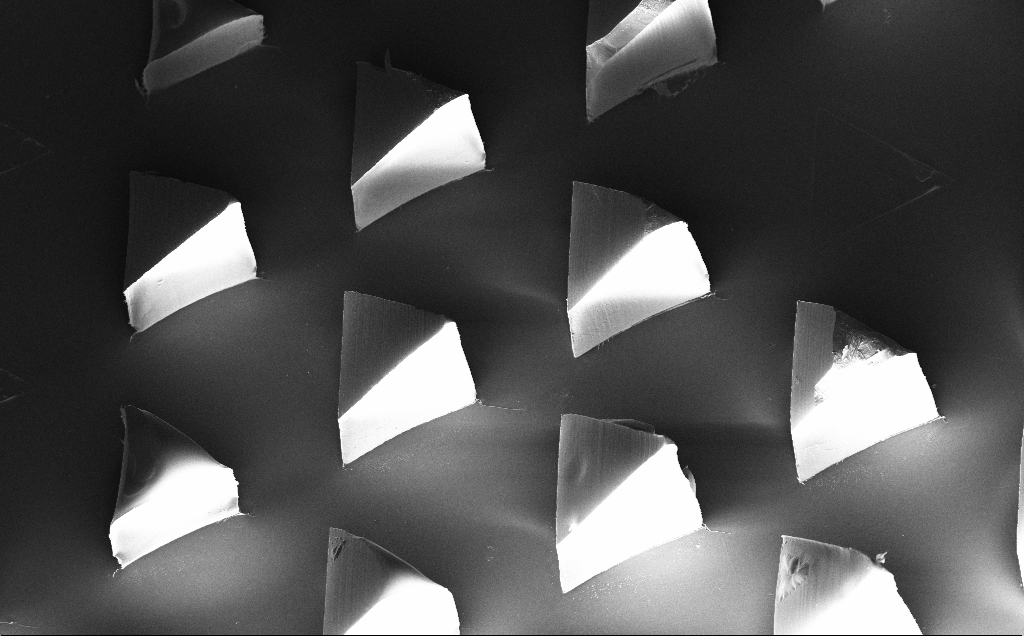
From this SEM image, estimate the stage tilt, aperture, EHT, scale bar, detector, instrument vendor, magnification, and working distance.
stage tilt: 20°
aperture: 30 µm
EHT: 10 kV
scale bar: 100000 nm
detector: InLens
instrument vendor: Zeiss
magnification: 0.212 K X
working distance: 11 mm